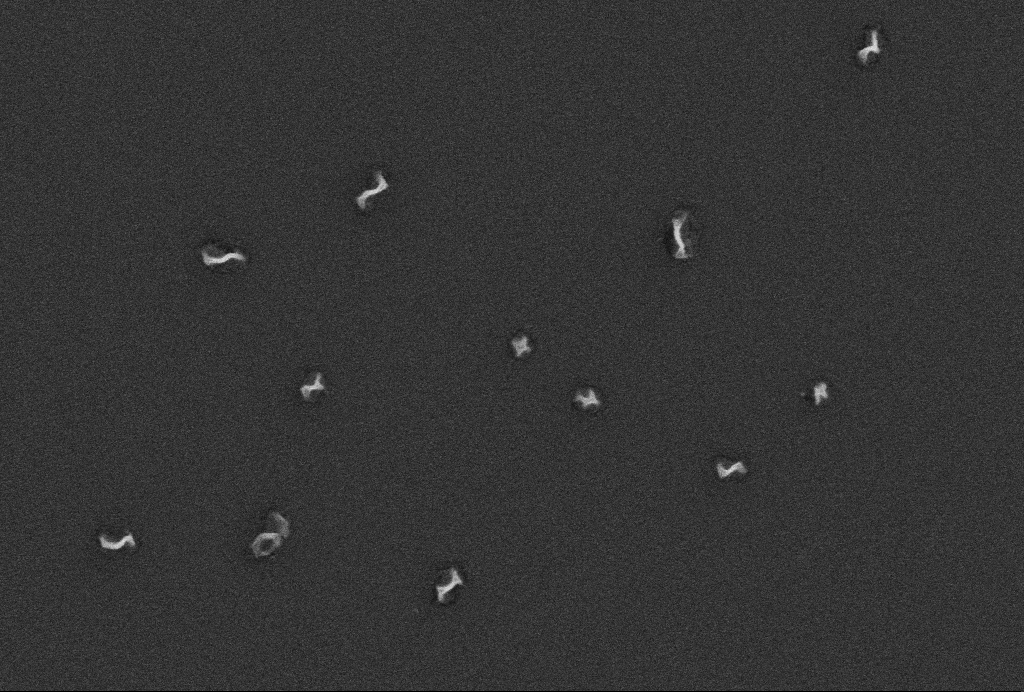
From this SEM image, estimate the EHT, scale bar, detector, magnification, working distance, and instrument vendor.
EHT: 5 kV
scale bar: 200 nm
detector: SE2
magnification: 100 K X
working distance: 2.6 mm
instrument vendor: Zeiss